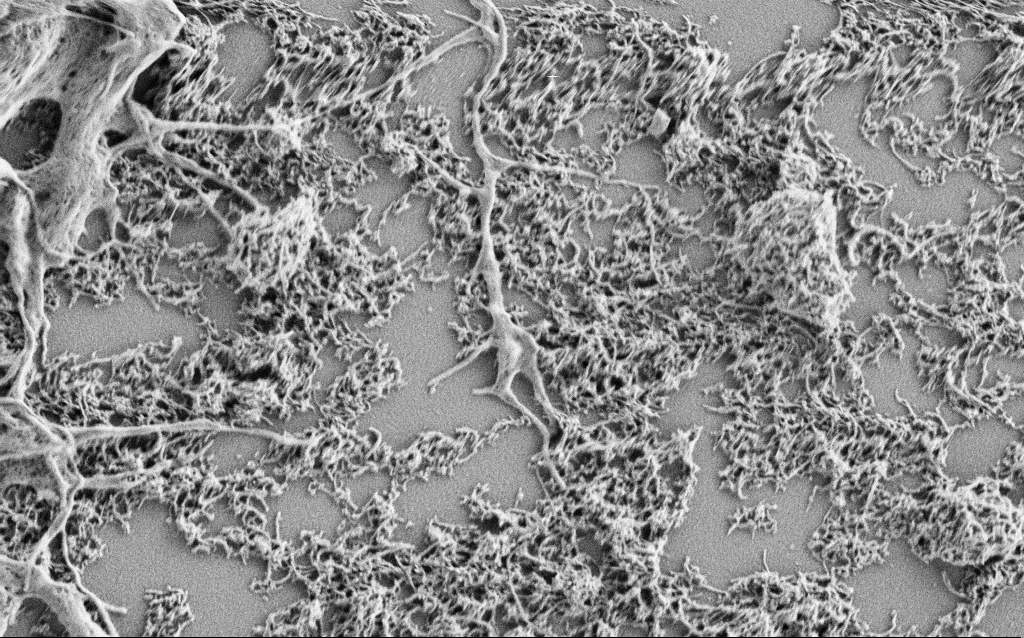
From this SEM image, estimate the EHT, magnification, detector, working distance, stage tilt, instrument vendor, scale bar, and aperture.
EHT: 1 kV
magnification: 20 K X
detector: SE2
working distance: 3 mm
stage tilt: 0°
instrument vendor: Zeiss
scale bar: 1000 nm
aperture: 30 µm